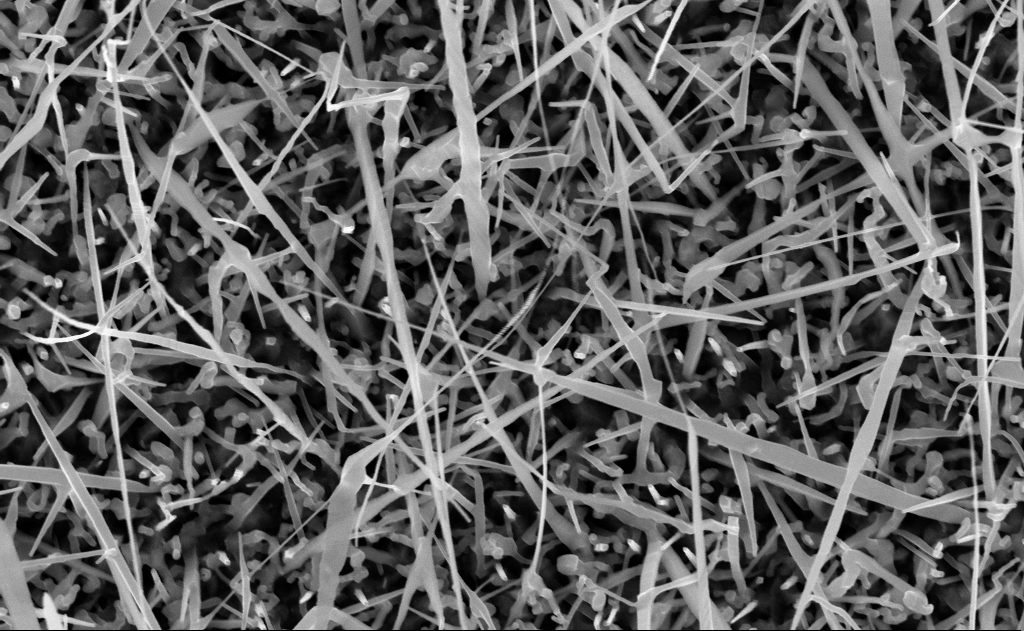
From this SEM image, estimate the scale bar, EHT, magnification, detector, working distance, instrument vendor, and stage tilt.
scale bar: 1000 nm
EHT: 10 kV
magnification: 40 K X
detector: InLens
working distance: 10 mm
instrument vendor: Zeiss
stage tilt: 0°